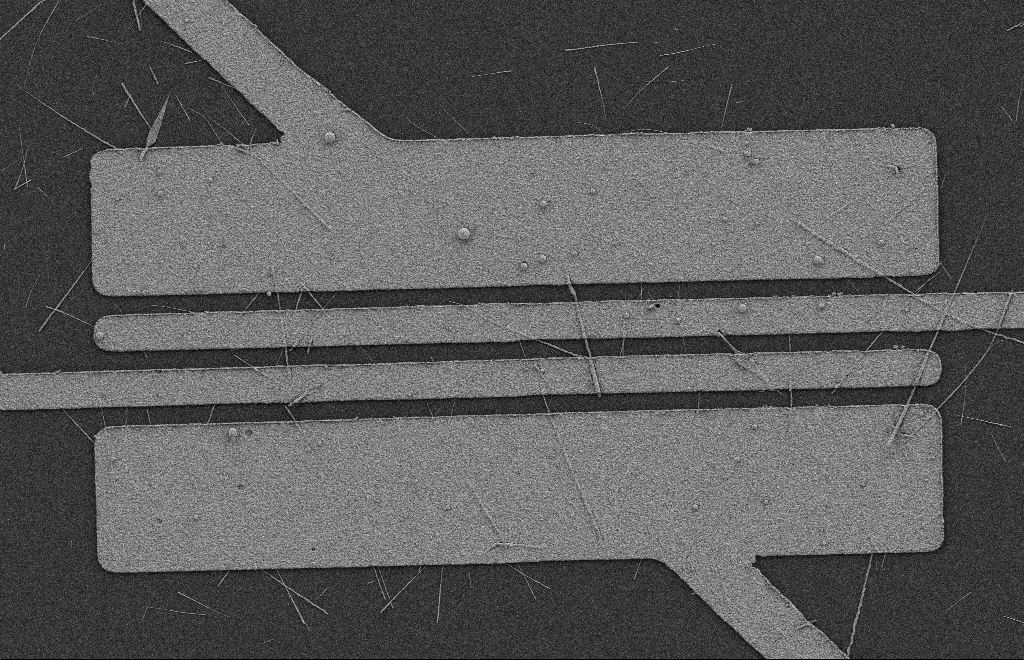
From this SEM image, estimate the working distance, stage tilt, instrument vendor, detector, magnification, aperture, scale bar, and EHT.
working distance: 9 mm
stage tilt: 0°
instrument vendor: Zeiss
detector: SE2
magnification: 5.08 K X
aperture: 20 µm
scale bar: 2000 nm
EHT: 2 kV